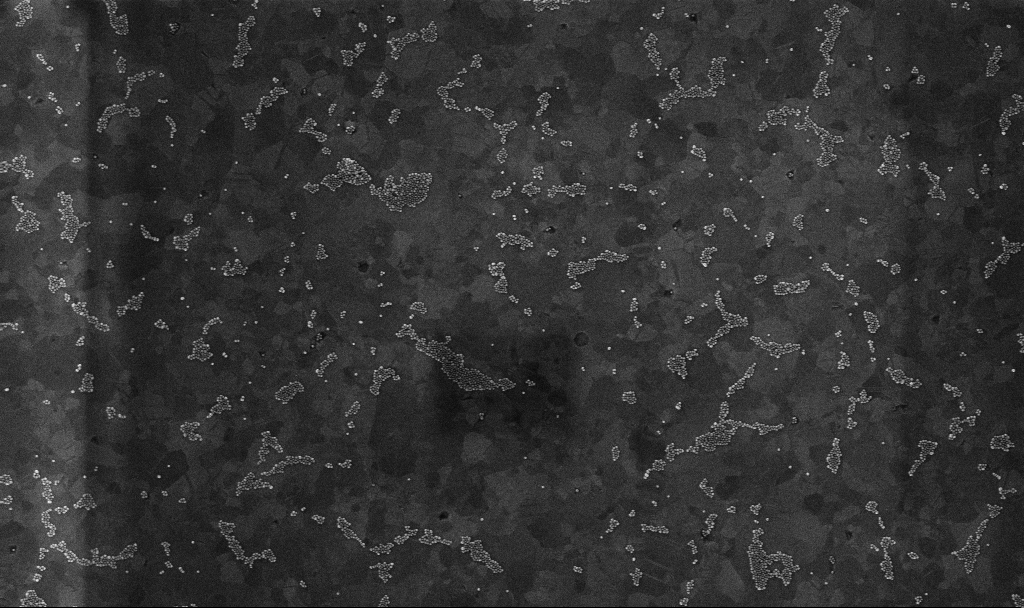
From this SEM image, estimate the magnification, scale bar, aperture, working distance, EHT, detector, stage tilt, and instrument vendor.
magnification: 50 K X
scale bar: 1000 nm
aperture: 30 µm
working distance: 3.2 mm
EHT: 10 kV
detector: InLens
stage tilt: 0°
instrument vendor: Zeiss